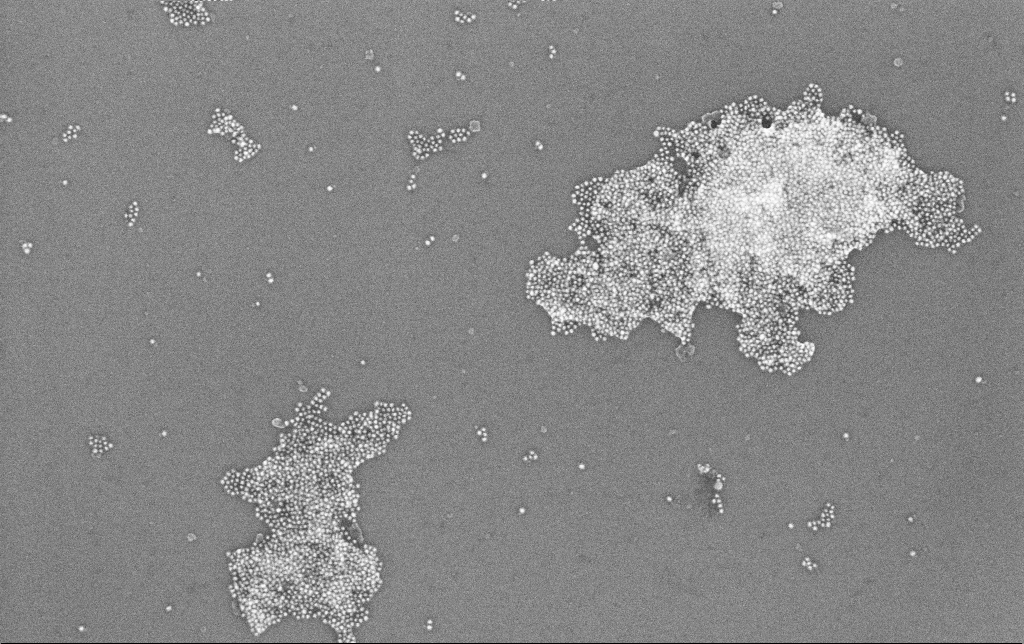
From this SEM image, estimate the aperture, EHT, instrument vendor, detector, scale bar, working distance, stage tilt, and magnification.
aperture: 30 µm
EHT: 10 kV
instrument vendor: Zeiss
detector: InLens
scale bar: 200 nm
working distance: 3.4 mm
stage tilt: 0°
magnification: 100 K X